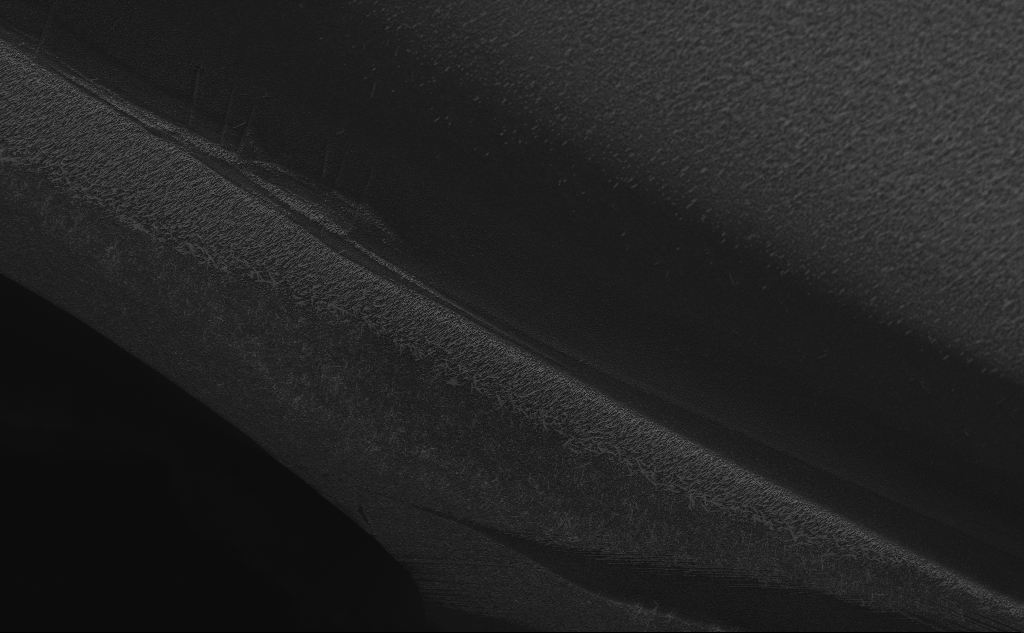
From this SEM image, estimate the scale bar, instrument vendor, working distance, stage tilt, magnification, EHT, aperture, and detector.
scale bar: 10000 nm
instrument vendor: Zeiss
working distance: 6 mm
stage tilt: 45°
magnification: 3.17 K X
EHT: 10 kV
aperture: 30 µm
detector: InLens